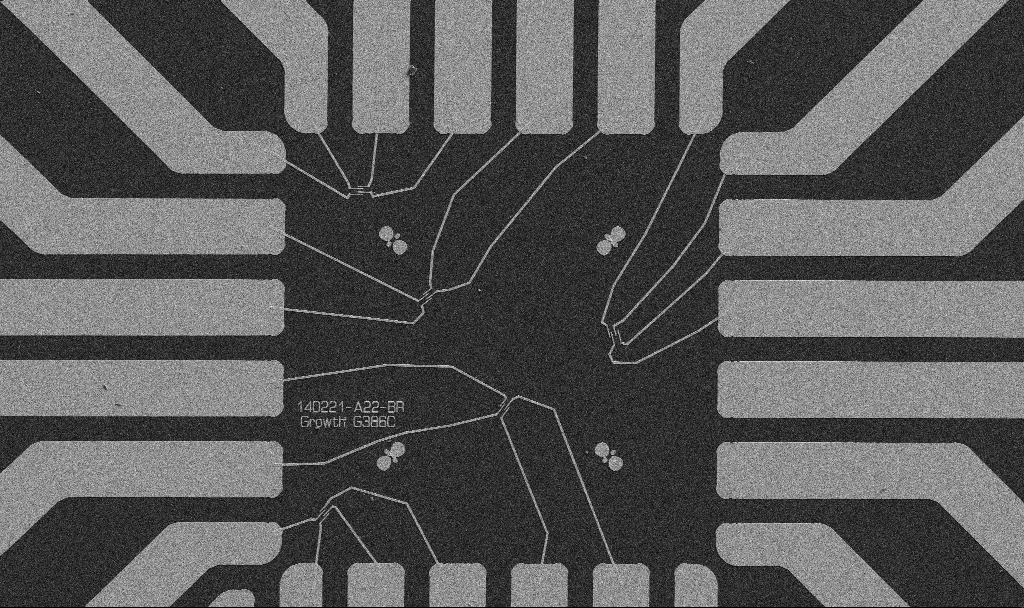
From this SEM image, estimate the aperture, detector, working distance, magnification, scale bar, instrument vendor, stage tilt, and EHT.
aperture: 30 µm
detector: SE2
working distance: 10.7 mm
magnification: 1 K X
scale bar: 20000 nm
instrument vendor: Zeiss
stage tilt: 0°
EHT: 5 kV